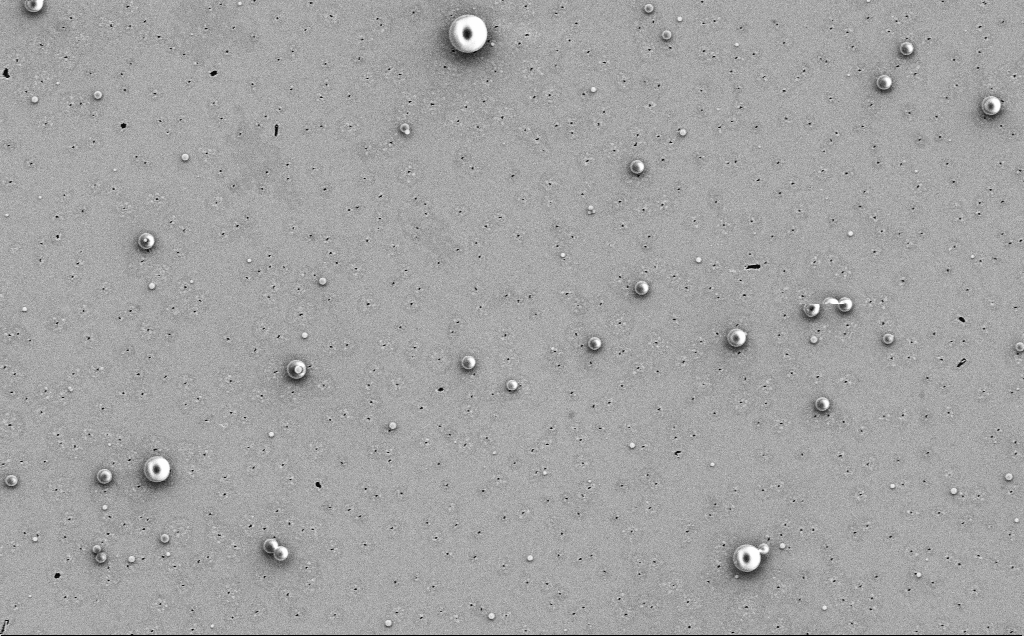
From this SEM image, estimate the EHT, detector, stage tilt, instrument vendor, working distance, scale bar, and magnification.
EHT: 5 kV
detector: SE2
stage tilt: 0°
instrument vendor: Zeiss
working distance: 12 mm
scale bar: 10000 nm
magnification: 4.2 K X